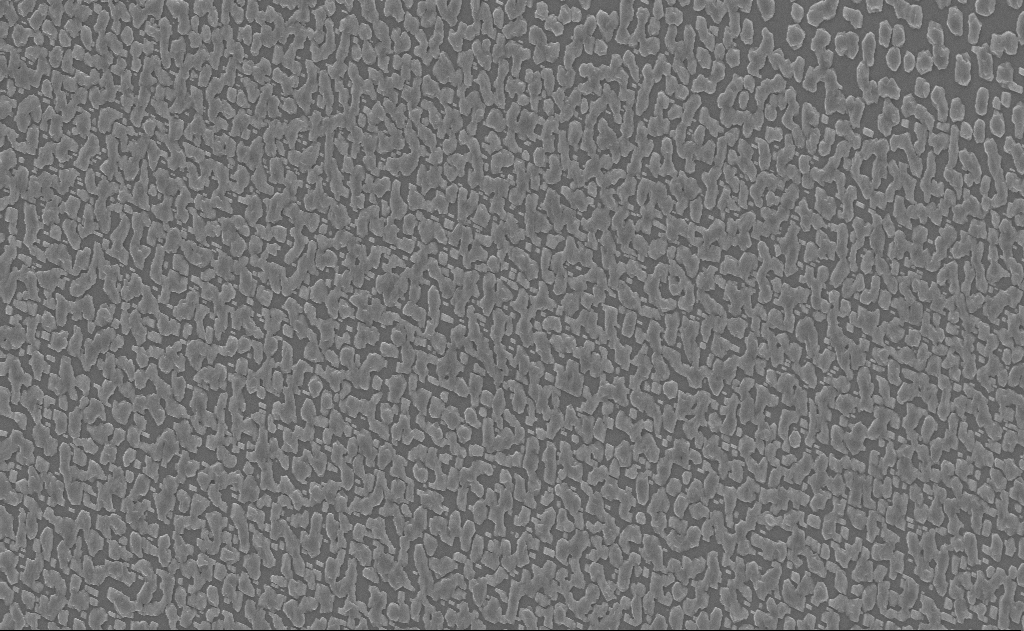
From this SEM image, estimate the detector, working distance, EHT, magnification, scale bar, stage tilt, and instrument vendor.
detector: InLens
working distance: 18 mm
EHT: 10 kV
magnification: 5 K X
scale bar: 10000 nm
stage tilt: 0°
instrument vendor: Zeiss